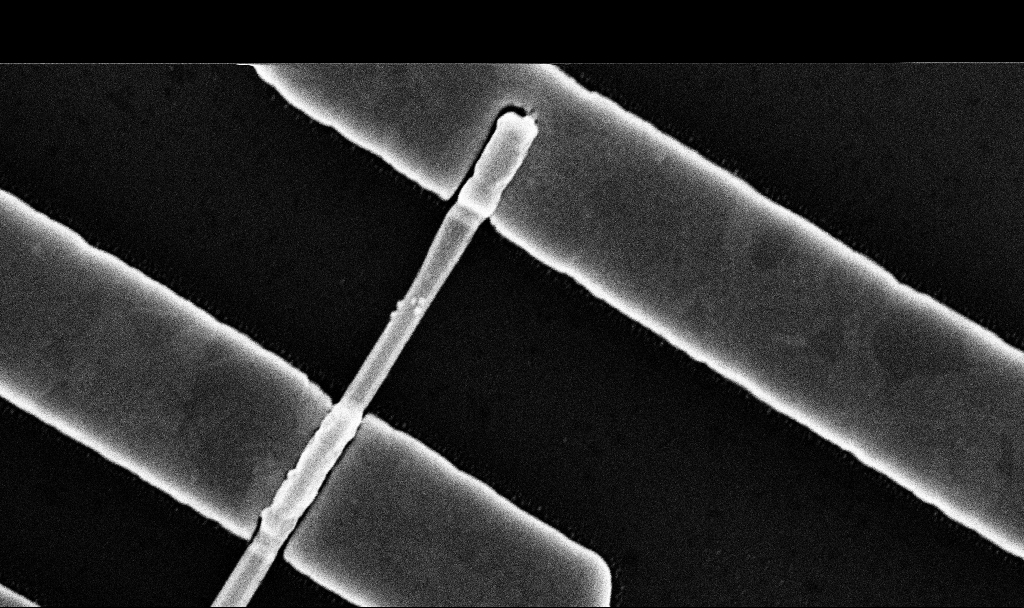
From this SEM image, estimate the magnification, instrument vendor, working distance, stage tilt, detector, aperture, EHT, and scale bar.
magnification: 88.53 K X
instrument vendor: Zeiss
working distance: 7.8 mm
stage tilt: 0°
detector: InLens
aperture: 30 µm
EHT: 10 kV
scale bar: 200 nm